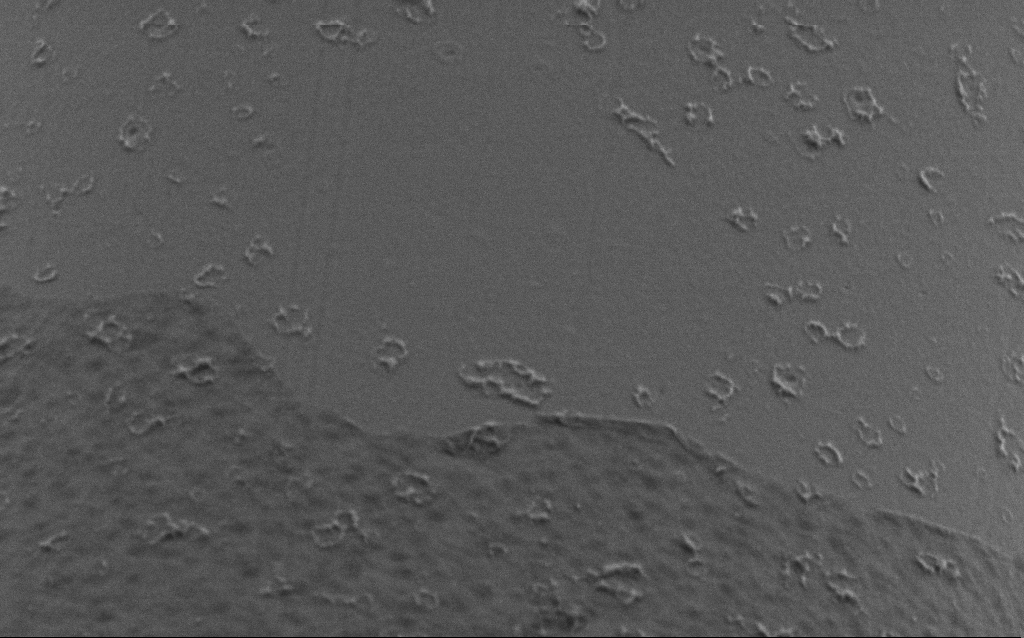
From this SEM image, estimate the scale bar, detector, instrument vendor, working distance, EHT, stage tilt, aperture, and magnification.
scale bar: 1000 nm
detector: SE2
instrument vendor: Zeiss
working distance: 6 mm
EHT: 1 kV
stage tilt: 45°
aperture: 30 µm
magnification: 50 K X